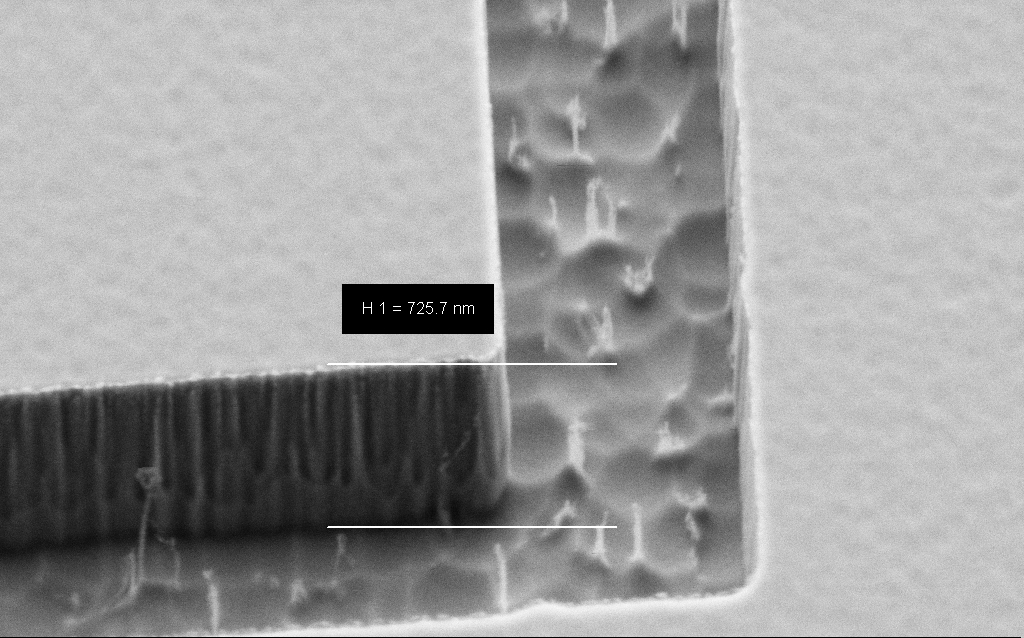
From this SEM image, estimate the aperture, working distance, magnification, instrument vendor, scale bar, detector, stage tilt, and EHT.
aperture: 30 µm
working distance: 6 mm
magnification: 82.48 K X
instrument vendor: Zeiss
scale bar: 200 nm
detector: SE2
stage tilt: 45°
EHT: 2 kV